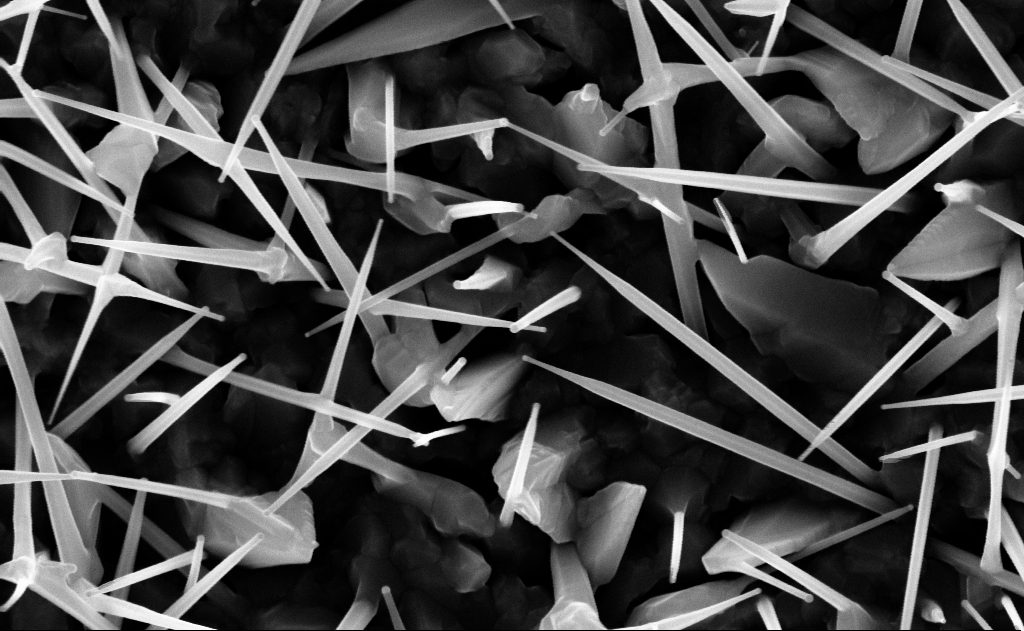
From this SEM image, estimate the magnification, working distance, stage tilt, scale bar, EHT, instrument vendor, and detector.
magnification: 80 K X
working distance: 9 mm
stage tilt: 0°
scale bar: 200 nm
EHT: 10 kV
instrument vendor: Zeiss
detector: InLens